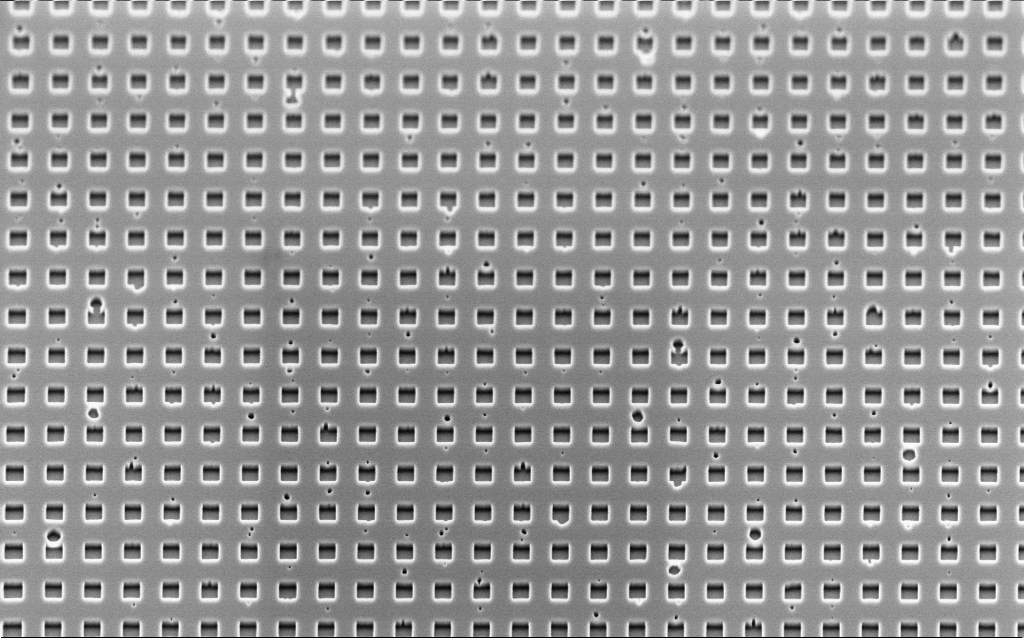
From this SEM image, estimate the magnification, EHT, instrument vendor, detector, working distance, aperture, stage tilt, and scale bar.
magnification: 28.73 K X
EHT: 2 kV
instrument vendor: Zeiss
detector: InLens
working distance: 3.3 mm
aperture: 30 µm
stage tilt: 45°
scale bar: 2000 nm